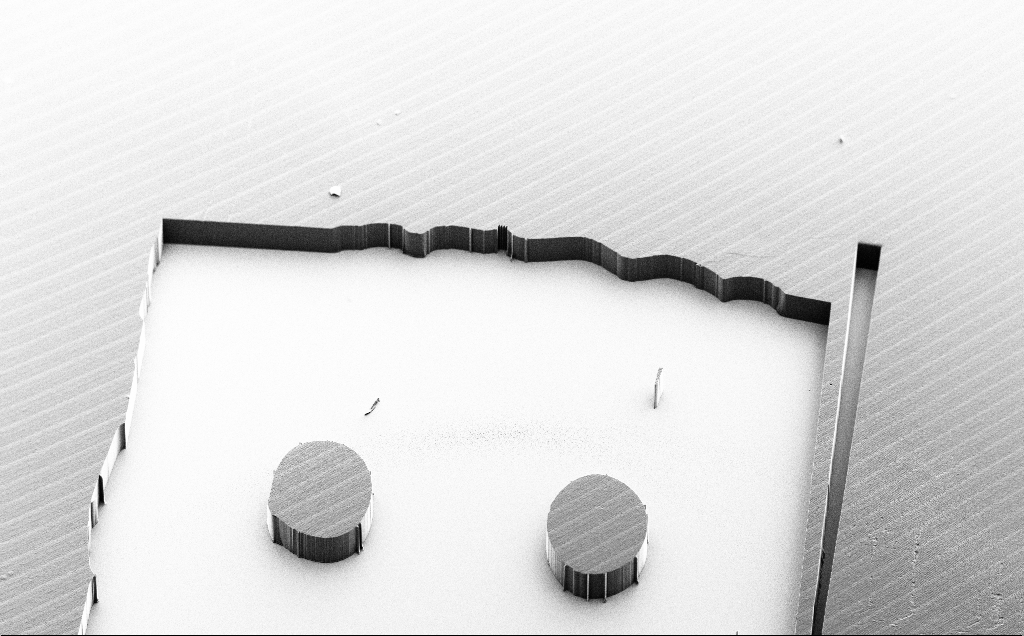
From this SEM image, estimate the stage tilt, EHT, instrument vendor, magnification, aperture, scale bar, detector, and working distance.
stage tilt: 45°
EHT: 10 kV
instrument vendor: Zeiss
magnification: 0.085 K X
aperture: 30 µm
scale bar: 200000 nm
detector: SE2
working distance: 11 mm